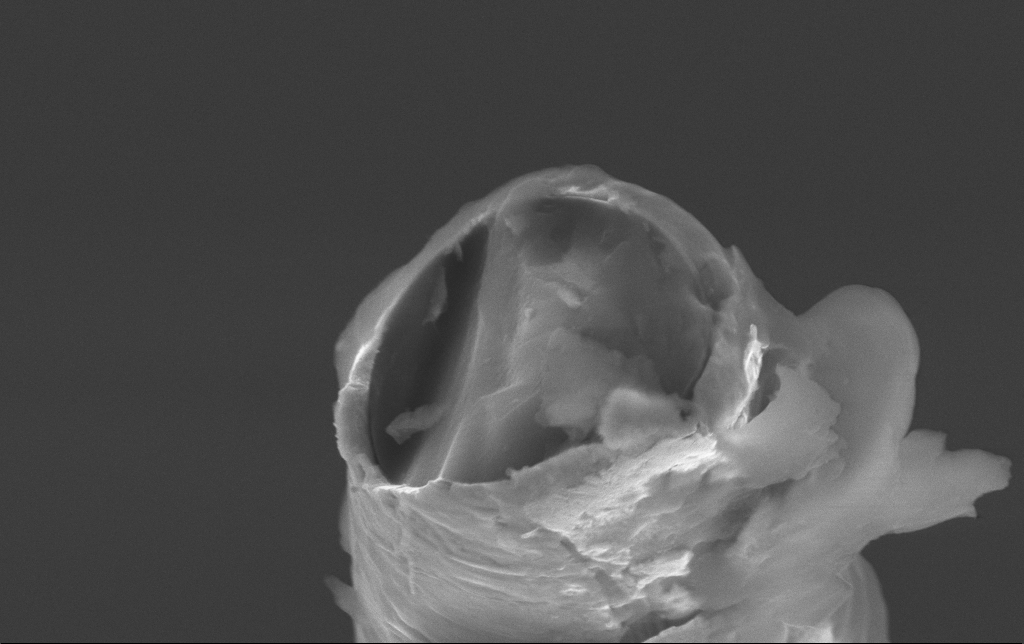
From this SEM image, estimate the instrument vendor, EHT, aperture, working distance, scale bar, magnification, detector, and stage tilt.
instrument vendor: Zeiss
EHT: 10 kV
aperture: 30 µm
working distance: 9.9 mm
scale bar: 2000 nm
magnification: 33.73 K X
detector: SE2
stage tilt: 69.3°